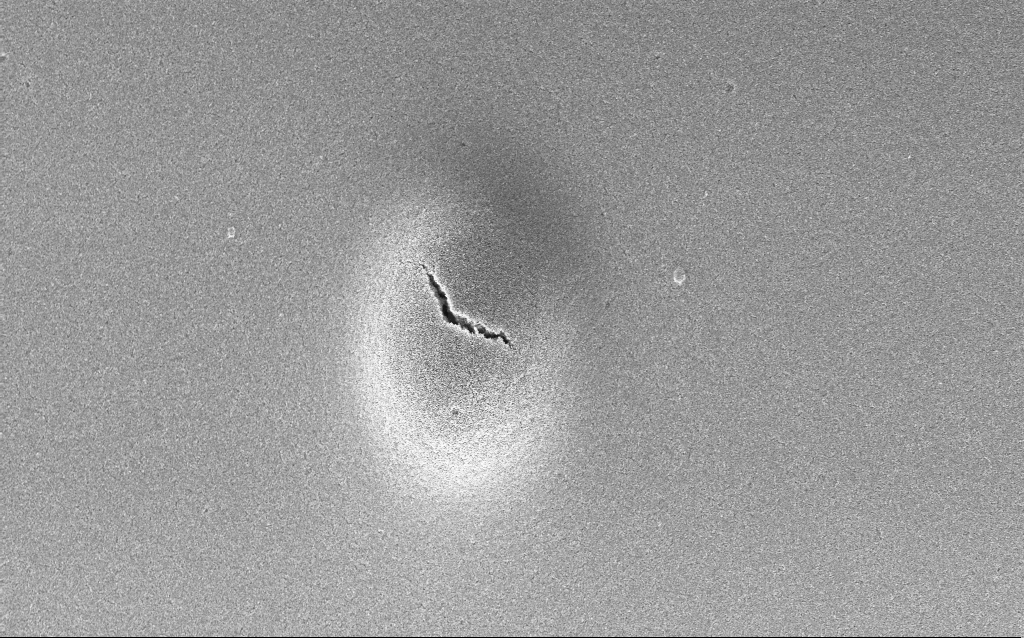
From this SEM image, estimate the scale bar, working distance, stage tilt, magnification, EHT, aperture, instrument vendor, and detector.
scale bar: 10000 nm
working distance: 2.6 mm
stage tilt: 0°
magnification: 5.83 K X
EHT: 5 kV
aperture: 30 µm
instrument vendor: Zeiss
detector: InLens